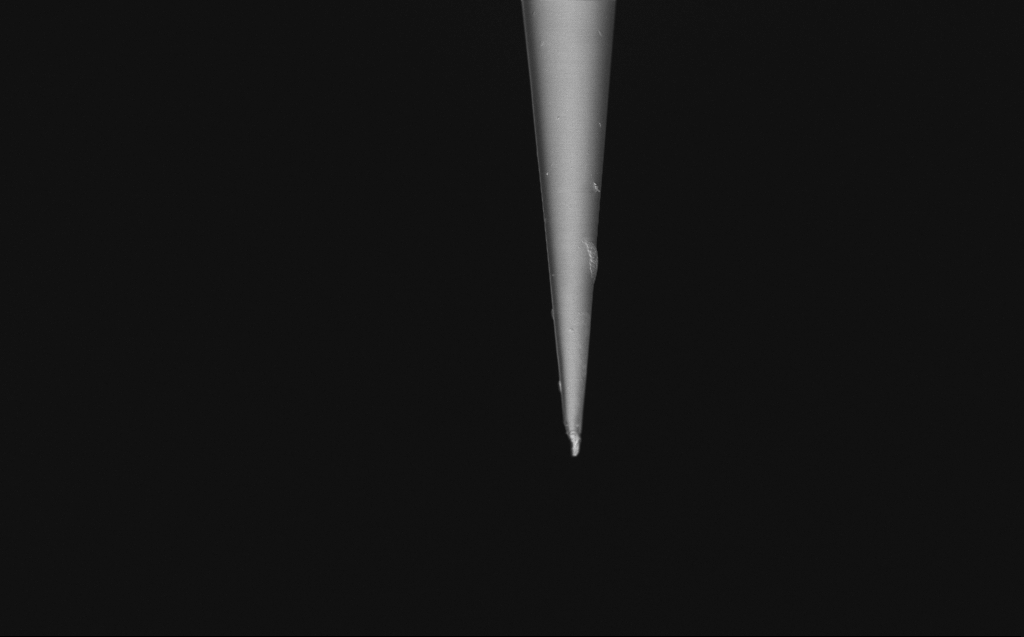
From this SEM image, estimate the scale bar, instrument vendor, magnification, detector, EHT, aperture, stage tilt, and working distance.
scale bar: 10000 nm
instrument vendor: Zeiss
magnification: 5 K X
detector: InLens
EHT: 1 kV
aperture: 30 µm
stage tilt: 45°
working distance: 5 mm